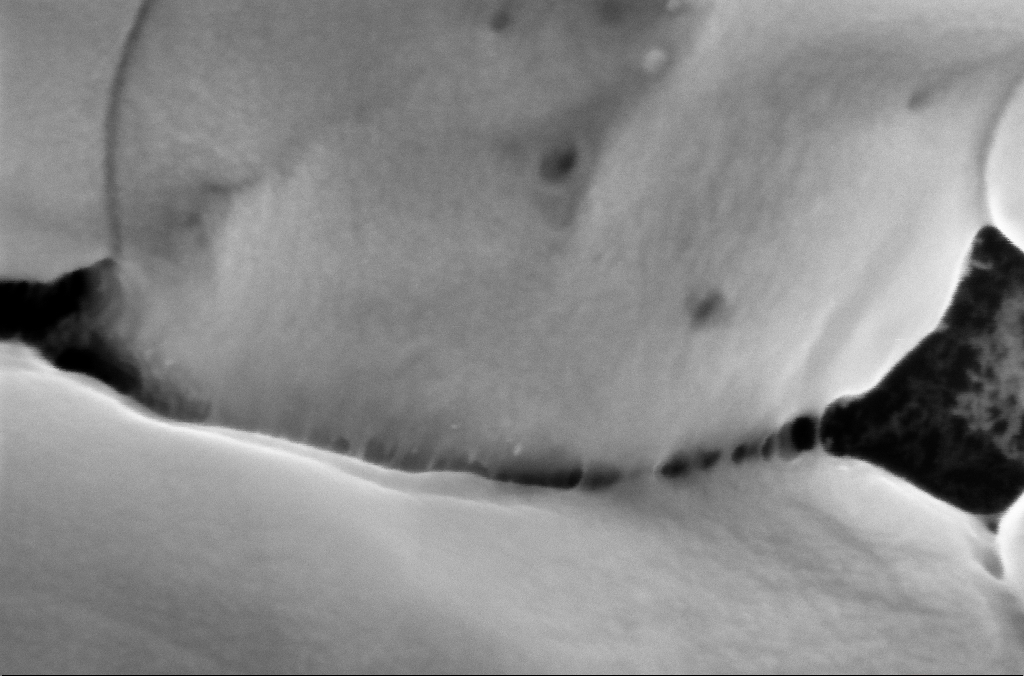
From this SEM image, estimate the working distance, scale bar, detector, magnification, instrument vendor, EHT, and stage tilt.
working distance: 3.1 mm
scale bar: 100 nm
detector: InLens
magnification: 392.66 K X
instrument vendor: Zeiss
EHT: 5 kV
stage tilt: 0°